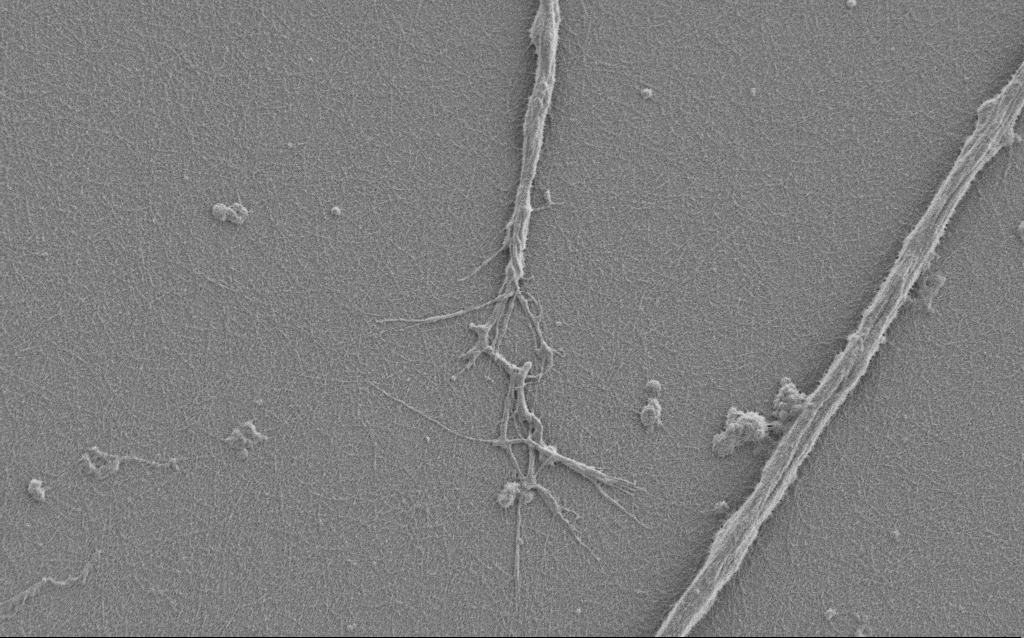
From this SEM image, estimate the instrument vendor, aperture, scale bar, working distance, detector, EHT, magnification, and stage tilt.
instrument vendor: Zeiss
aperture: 30 µm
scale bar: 2000 nm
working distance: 6 mm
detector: SE2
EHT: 1 kV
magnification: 7.5 K X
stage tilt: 0°